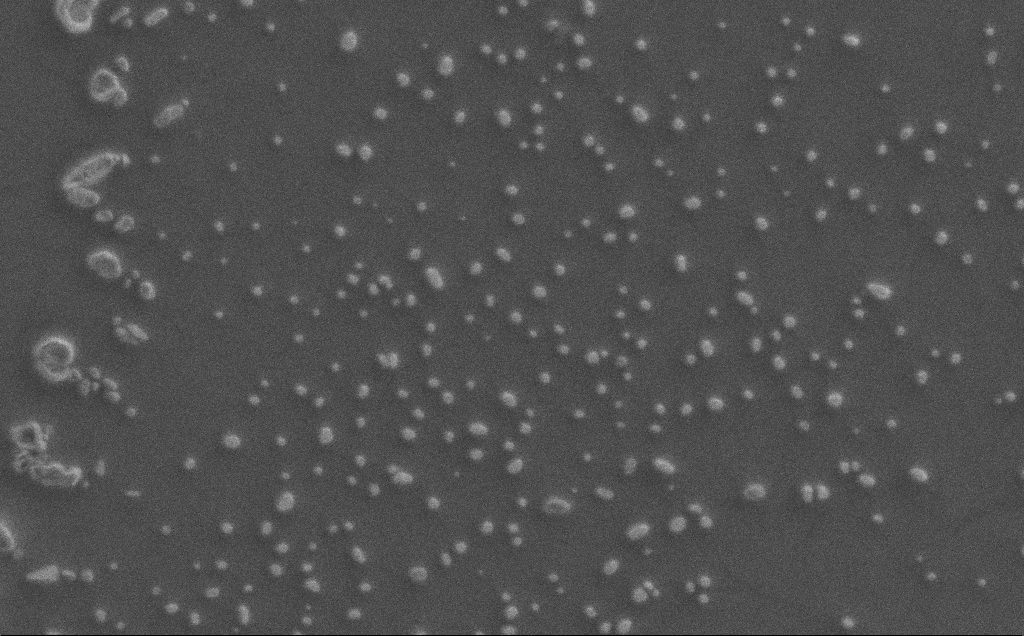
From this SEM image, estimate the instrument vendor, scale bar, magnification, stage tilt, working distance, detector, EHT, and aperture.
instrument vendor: Zeiss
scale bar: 2000 nm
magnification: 10.03 K X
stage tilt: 0°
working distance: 3 mm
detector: SE2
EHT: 0.5 kV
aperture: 30 µm